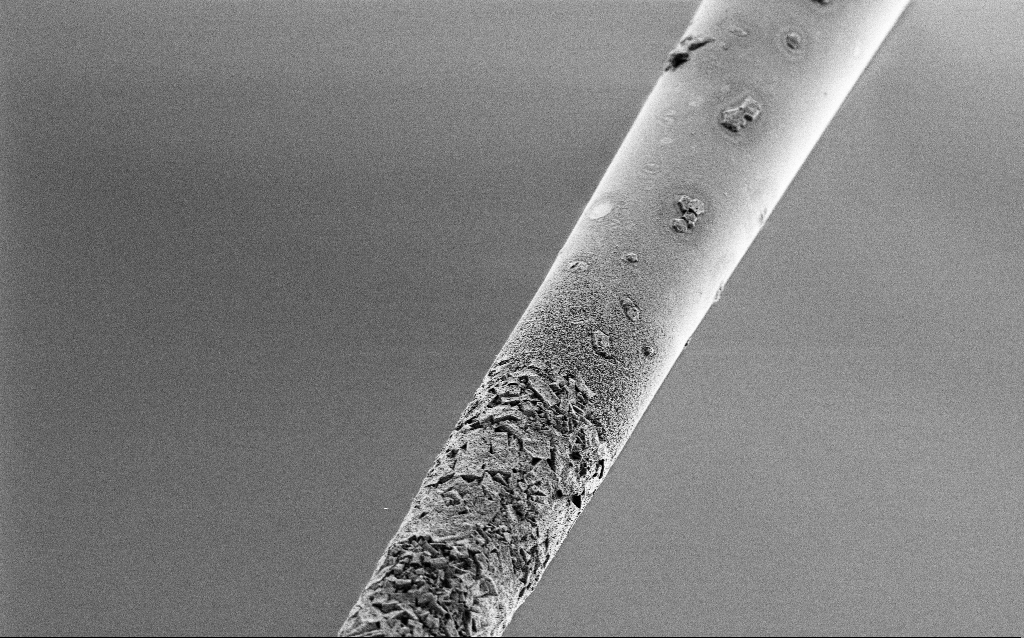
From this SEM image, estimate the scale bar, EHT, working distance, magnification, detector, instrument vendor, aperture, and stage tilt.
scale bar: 10000 nm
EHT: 1 kV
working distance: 6.8 mm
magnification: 2.5 K X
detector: SE2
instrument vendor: Zeiss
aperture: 30 µm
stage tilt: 45°